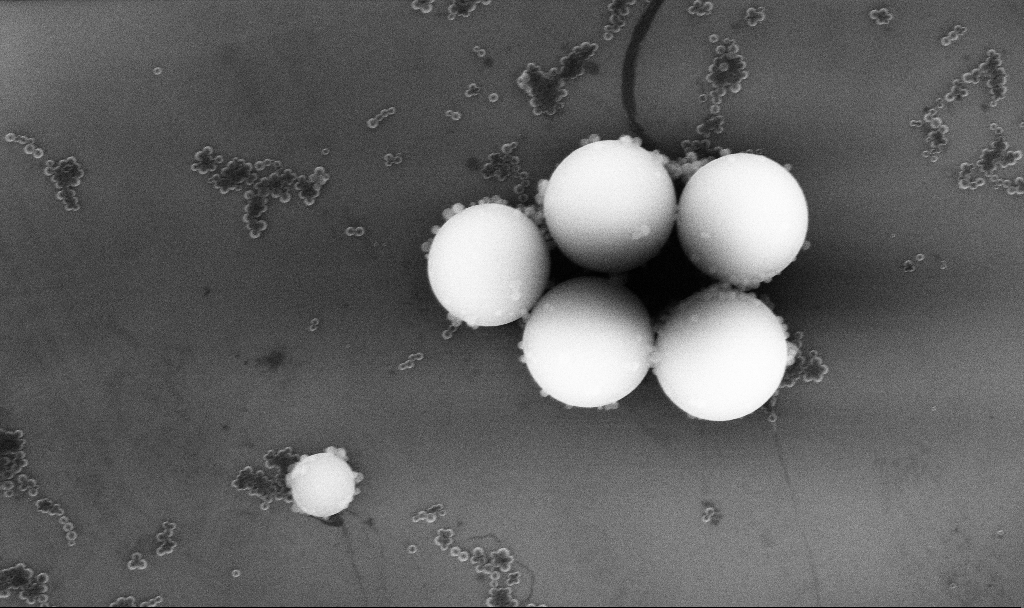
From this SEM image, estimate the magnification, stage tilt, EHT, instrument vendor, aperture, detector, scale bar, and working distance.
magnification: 23.56 K X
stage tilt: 0°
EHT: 10 kV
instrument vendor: Zeiss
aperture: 30 µm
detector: InLens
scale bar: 2000 nm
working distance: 5.2 mm